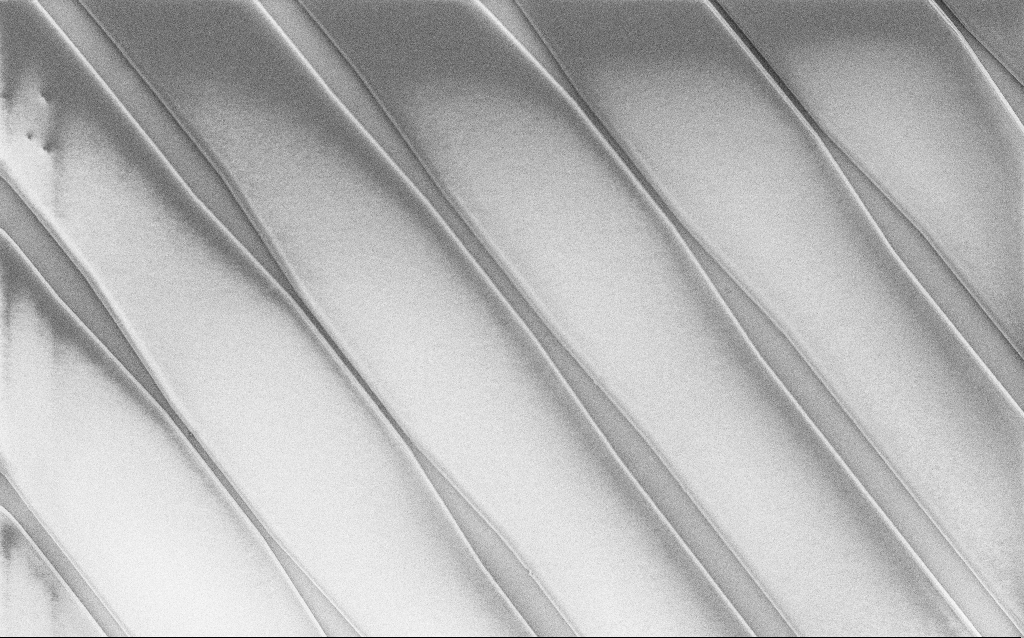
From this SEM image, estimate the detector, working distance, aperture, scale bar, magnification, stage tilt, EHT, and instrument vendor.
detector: SE2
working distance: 7 mm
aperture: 30 µm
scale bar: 100000 nm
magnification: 0.6 K X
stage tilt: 0°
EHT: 1 kV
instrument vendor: Zeiss